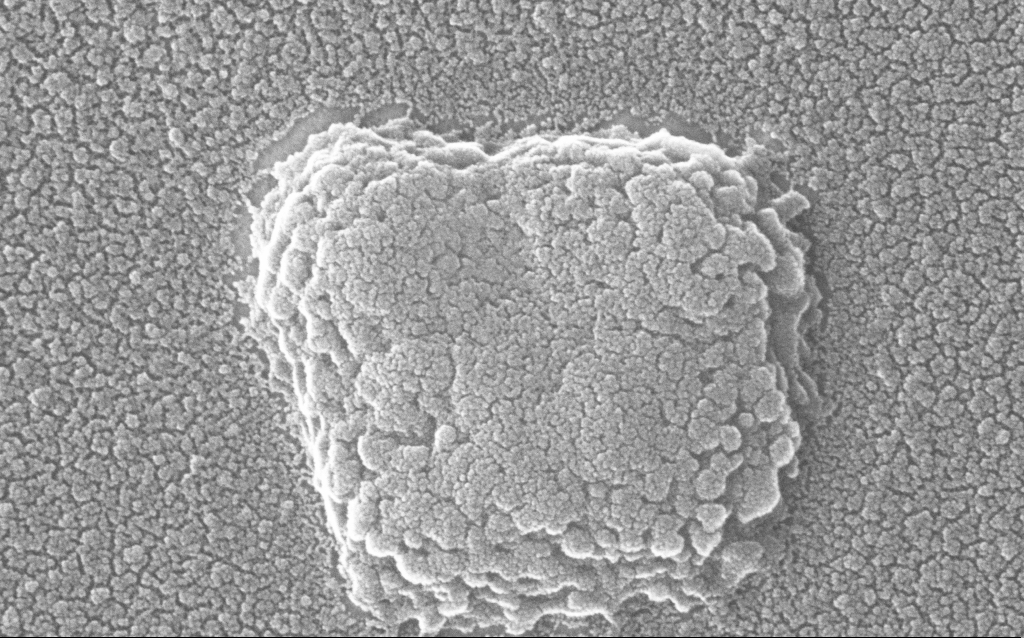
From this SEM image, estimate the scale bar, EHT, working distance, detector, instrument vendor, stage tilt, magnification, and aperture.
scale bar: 100 nm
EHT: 20 kV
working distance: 1.8 mm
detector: InLens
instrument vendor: Zeiss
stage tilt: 0°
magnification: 300 K X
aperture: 30 µm